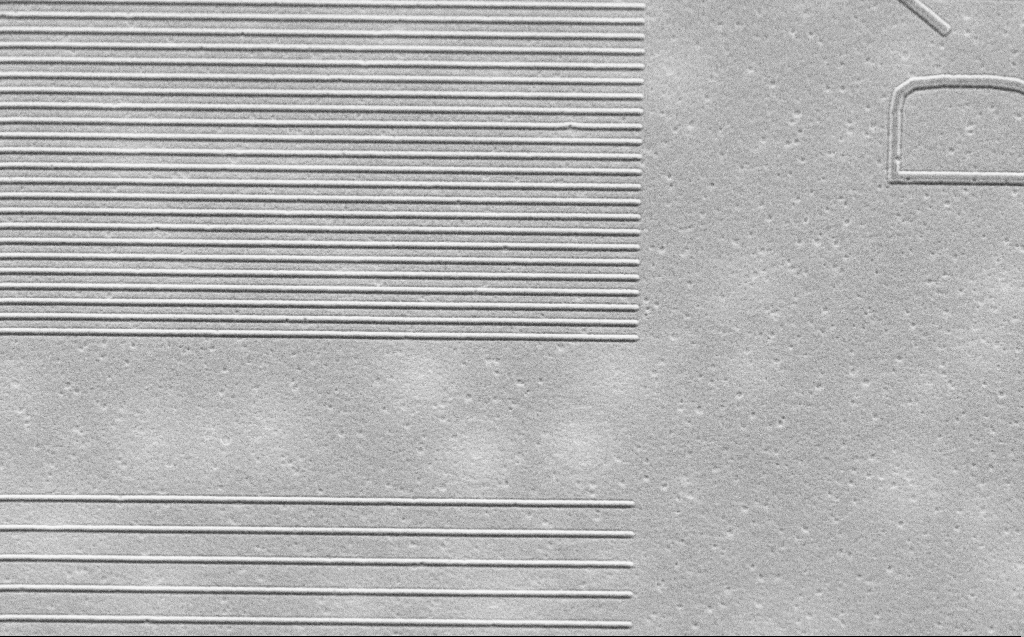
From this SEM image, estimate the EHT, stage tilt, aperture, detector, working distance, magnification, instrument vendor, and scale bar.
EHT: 2.5 kV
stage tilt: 30°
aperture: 30 µm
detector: SE2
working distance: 4 mm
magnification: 11.29 K X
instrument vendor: Zeiss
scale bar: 2000 nm